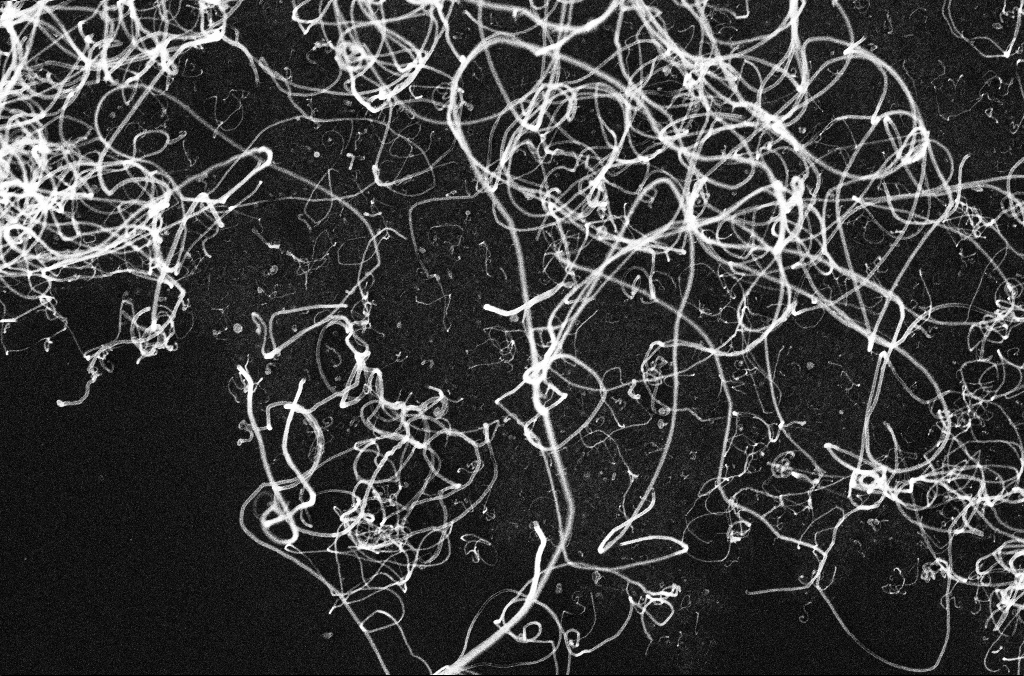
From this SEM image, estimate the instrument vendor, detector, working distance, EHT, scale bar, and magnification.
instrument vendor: Zeiss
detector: InLens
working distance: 3.3 mm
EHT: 10 kV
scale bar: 1000 nm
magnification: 50 K X